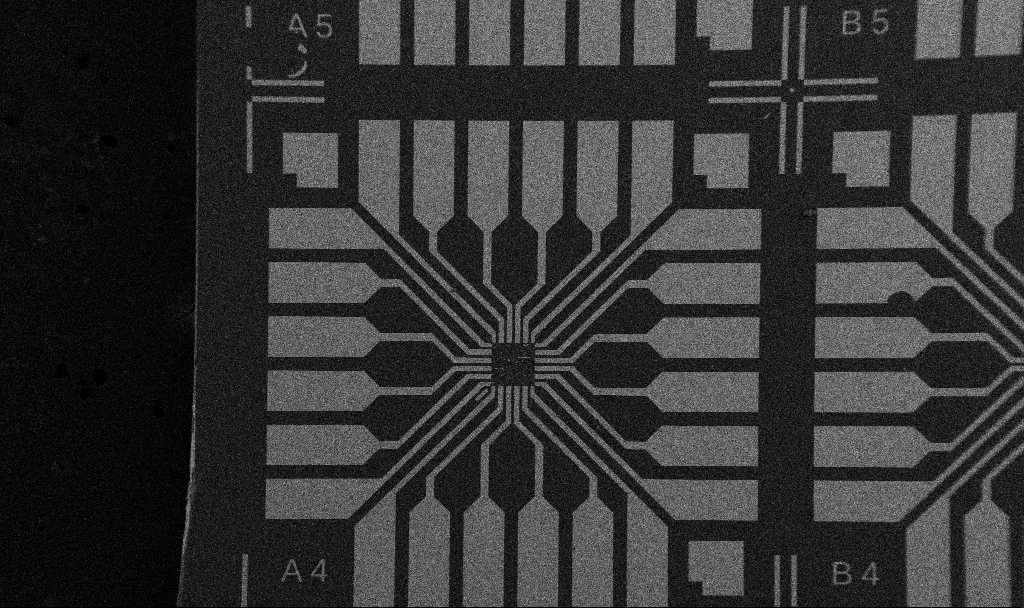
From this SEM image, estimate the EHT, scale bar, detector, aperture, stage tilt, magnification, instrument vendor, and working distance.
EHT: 5 kV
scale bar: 200000 nm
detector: SE2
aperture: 30 µm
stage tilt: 0°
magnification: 0.1 K X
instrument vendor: Zeiss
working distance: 10.7 mm